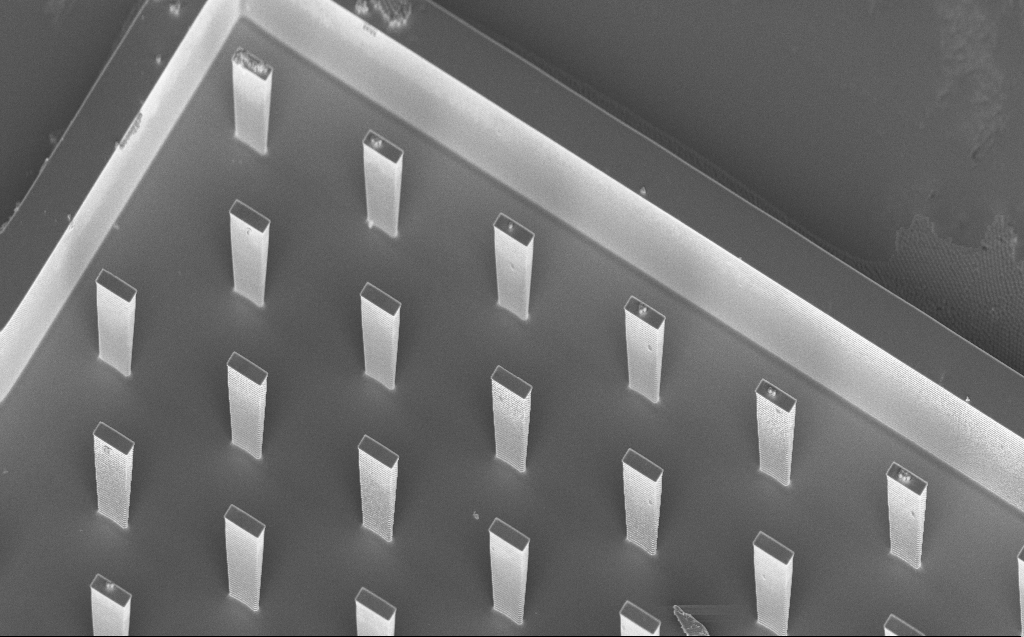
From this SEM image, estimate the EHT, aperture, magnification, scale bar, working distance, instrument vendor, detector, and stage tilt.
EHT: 5 kV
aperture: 30 µm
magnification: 1.99 K X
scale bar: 10000 nm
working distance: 7 mm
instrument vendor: Zeiss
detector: InLens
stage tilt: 45°